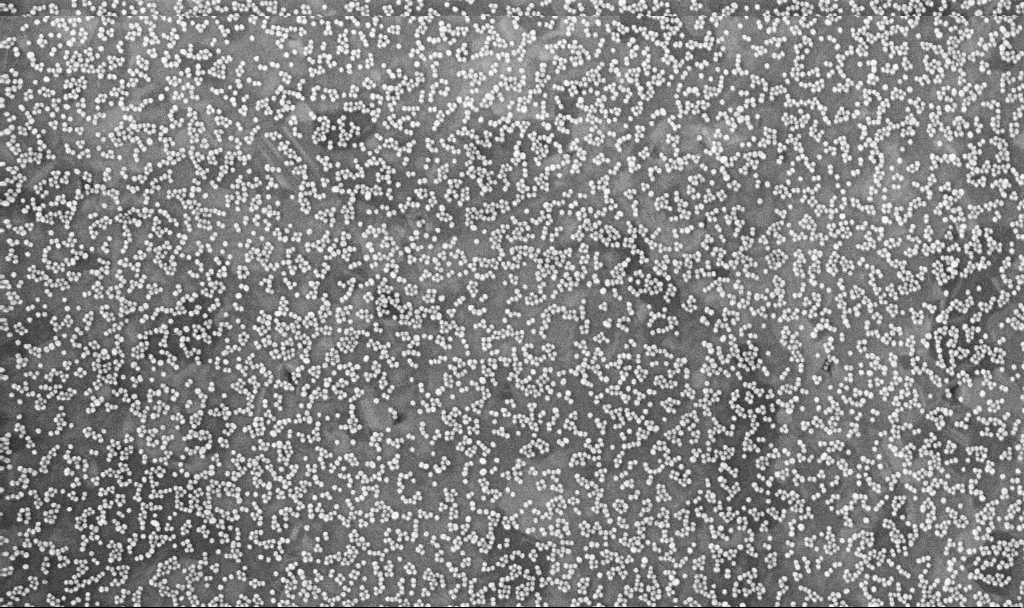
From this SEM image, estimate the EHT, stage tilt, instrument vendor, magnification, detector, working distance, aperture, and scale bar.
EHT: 10 kV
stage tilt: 0°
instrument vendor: Zeiss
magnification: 100 K X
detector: InLens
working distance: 3.3 mm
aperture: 30 µm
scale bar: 200 nm